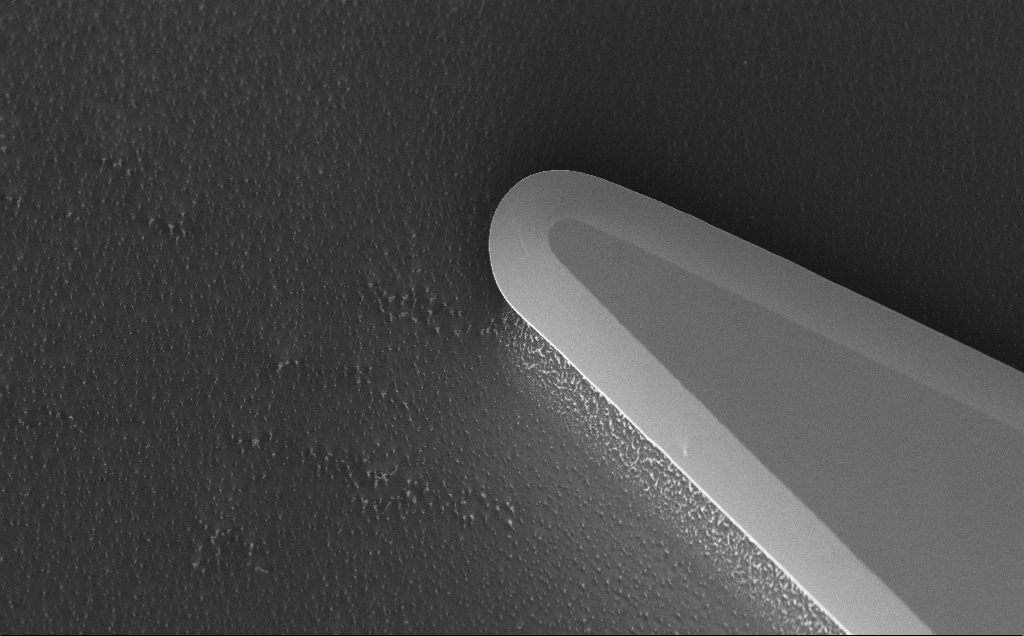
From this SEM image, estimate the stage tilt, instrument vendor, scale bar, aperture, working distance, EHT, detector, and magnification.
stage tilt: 45°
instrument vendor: Zeiss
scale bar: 20000 nm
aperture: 30 µm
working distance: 8 mm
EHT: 5 kV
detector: InLens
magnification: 2.9 K X